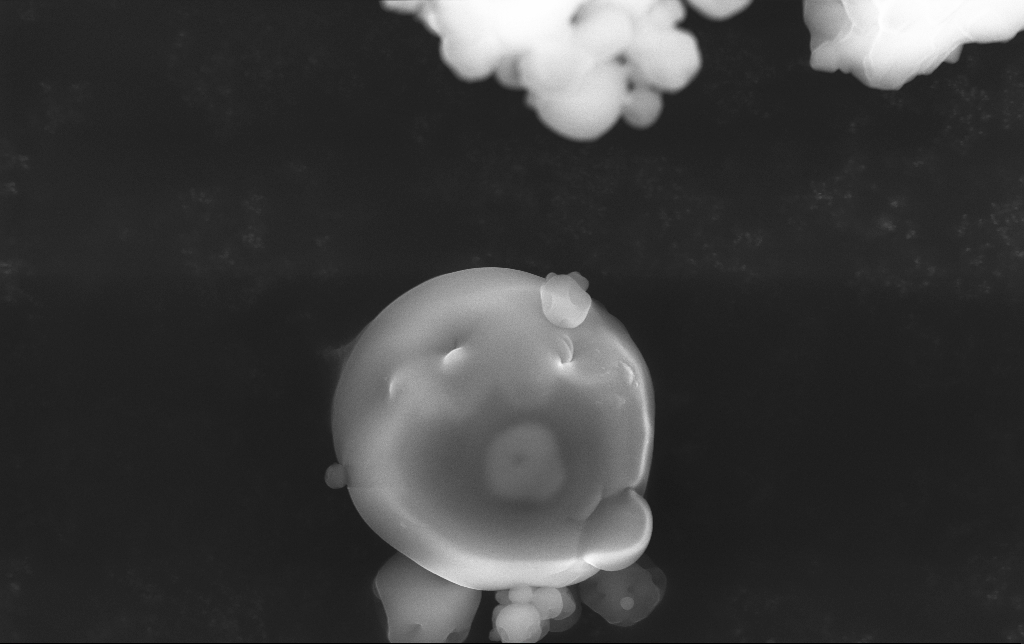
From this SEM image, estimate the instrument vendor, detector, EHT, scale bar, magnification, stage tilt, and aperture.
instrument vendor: Zeiss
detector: InLens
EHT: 15 kV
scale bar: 2000 nm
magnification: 15.88 K X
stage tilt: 0°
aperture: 30 µm